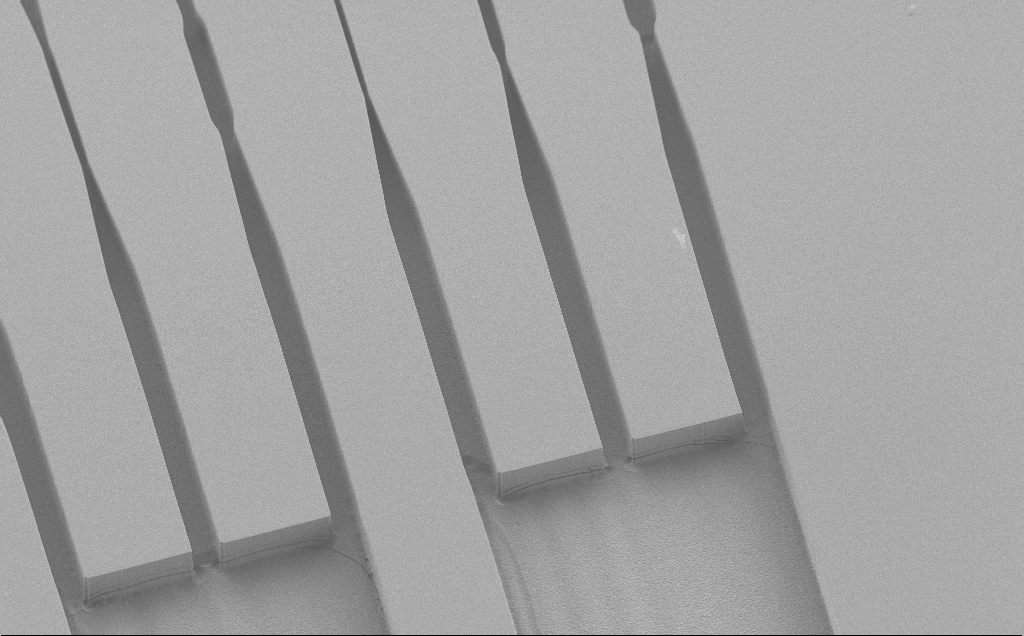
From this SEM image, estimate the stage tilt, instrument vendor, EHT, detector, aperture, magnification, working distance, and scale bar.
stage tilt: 30°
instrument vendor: Zeiss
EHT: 1.2 kV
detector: SE2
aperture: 30 µm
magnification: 0.491 K X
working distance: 6 mm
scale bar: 100000 nm